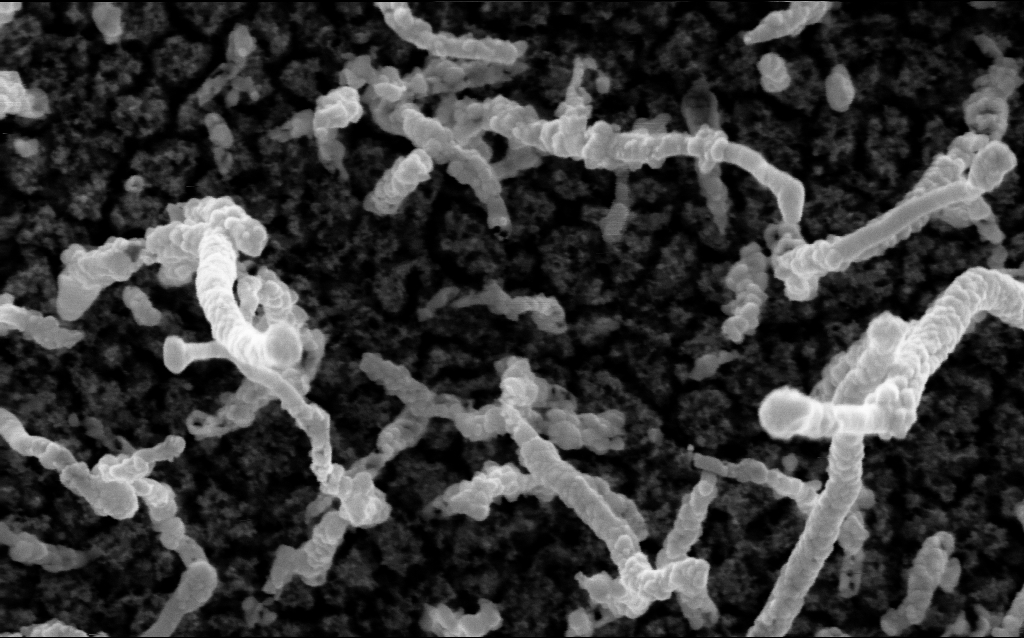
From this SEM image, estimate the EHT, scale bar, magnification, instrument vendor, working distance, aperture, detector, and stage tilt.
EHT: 5 kV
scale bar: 100 nm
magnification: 200 K X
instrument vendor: Zeiss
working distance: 2.1 mm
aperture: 30 µm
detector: InLens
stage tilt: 0°